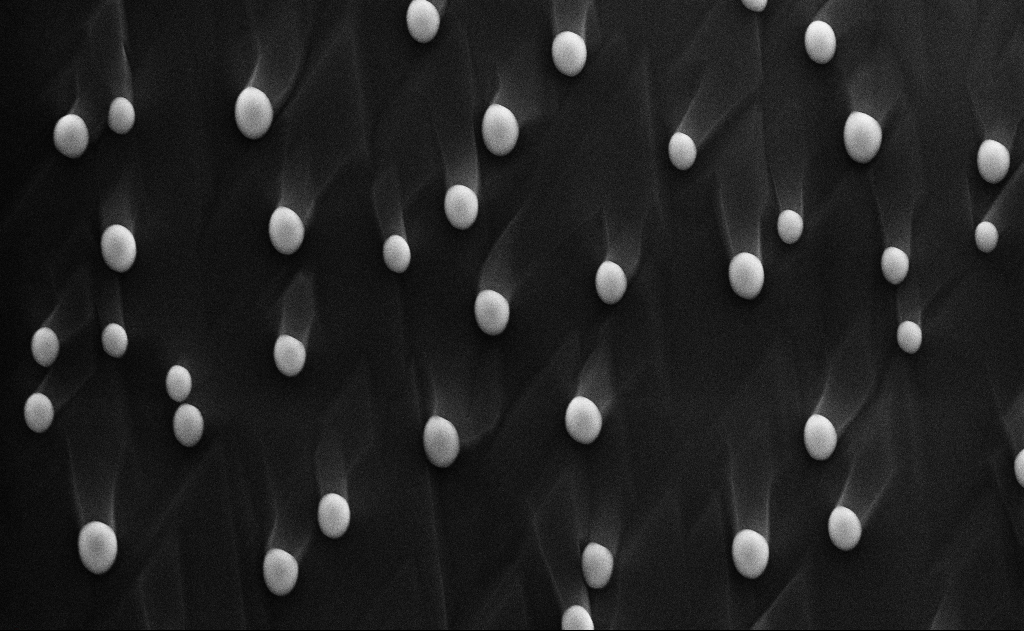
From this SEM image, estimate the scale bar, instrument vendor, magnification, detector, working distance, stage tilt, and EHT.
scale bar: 2000 nm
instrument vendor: Zeiss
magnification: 20 K X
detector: InLens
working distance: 12 mm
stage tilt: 0°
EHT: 10 kV